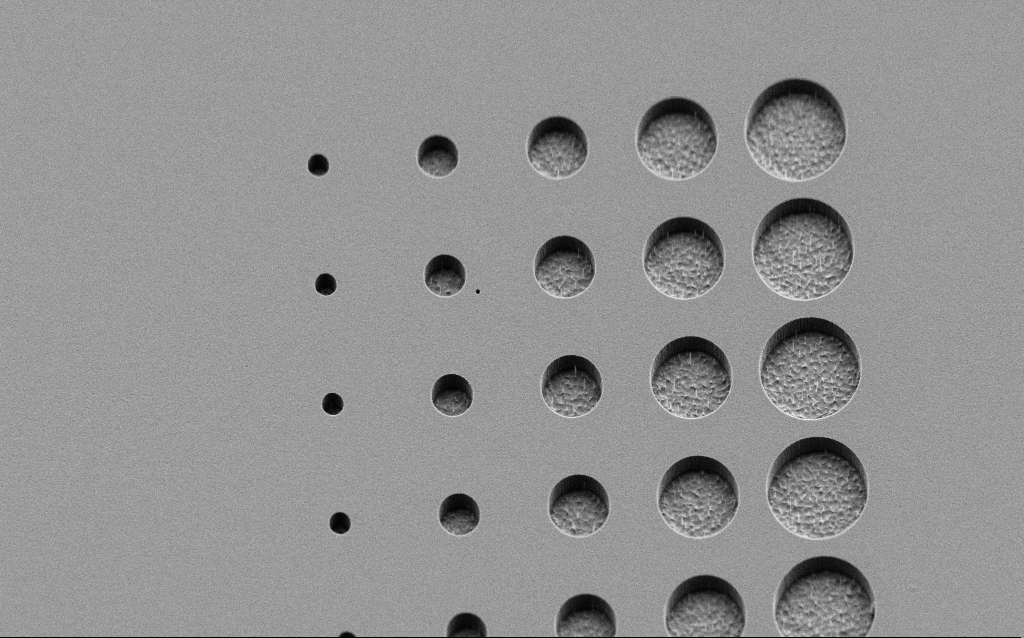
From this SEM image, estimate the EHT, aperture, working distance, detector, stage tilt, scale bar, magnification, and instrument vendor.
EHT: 2 kV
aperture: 30 µm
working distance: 6 mm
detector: SE2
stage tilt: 45°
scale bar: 2000 nm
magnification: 7.37 K X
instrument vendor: Zeiss